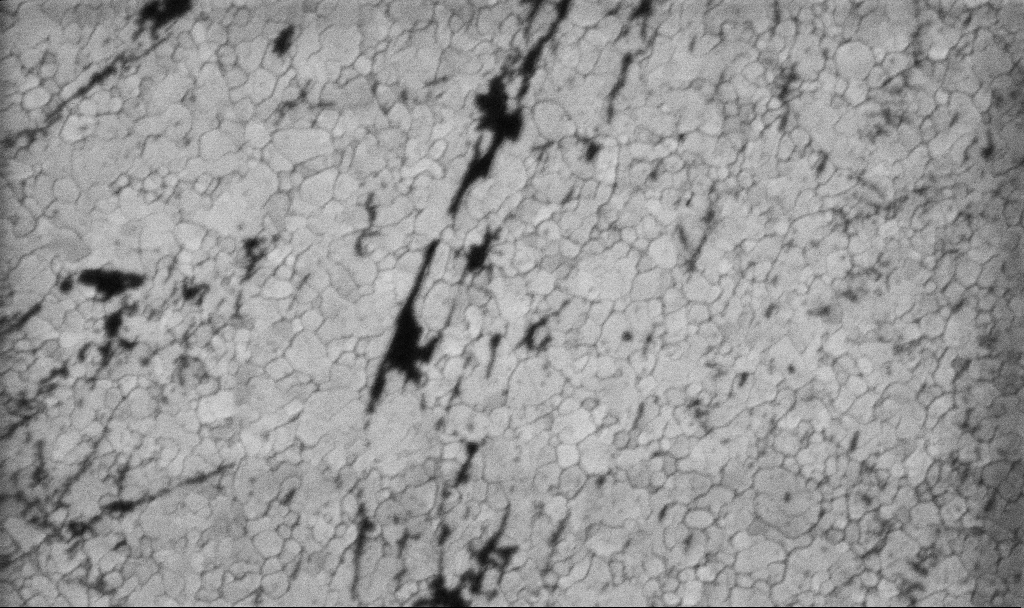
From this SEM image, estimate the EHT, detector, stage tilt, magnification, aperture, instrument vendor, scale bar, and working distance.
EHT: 5 kV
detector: InLens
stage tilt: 0°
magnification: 100.15 K X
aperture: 30 µm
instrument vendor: Zeiss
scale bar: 200 nm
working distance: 3.1 mm